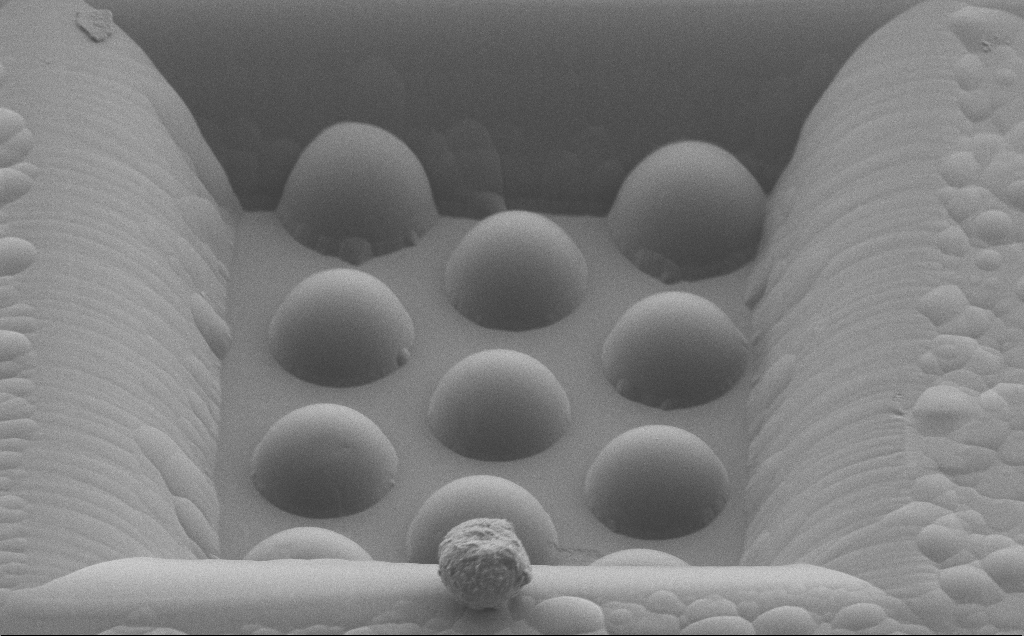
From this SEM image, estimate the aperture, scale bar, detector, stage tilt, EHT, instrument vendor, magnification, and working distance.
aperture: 30 µm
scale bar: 10000 nm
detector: SE2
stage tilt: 45°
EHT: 1.3 kV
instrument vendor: Zeiss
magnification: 3.38 K X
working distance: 7 mm